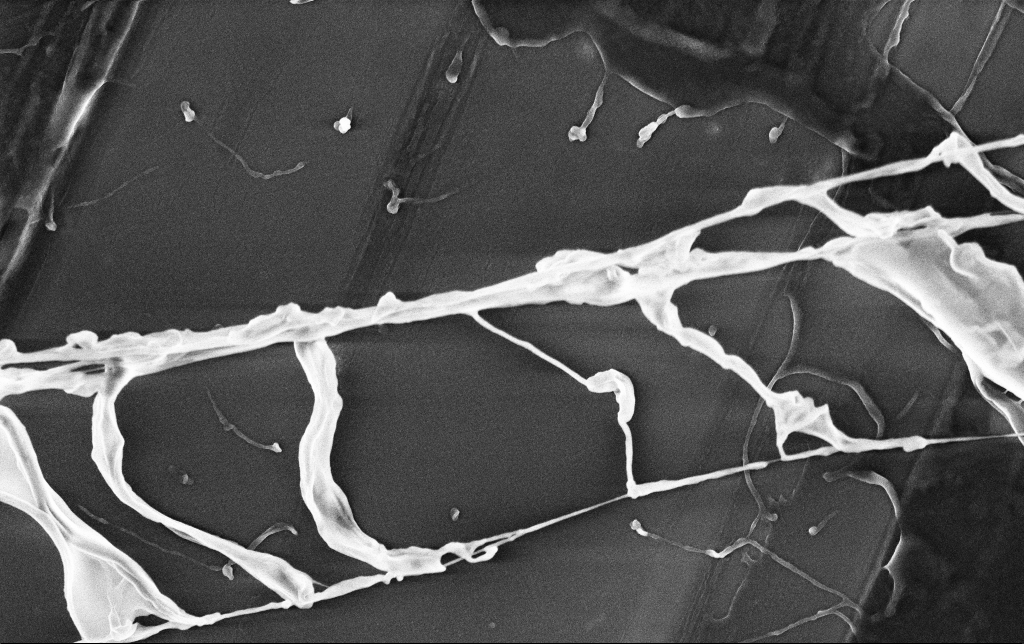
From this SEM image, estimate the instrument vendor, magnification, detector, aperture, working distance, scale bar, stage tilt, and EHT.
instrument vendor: Zeiss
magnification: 23.28 K X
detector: InLens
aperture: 30 µm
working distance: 3.5 mm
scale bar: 1000 nm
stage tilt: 0°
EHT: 3 kV